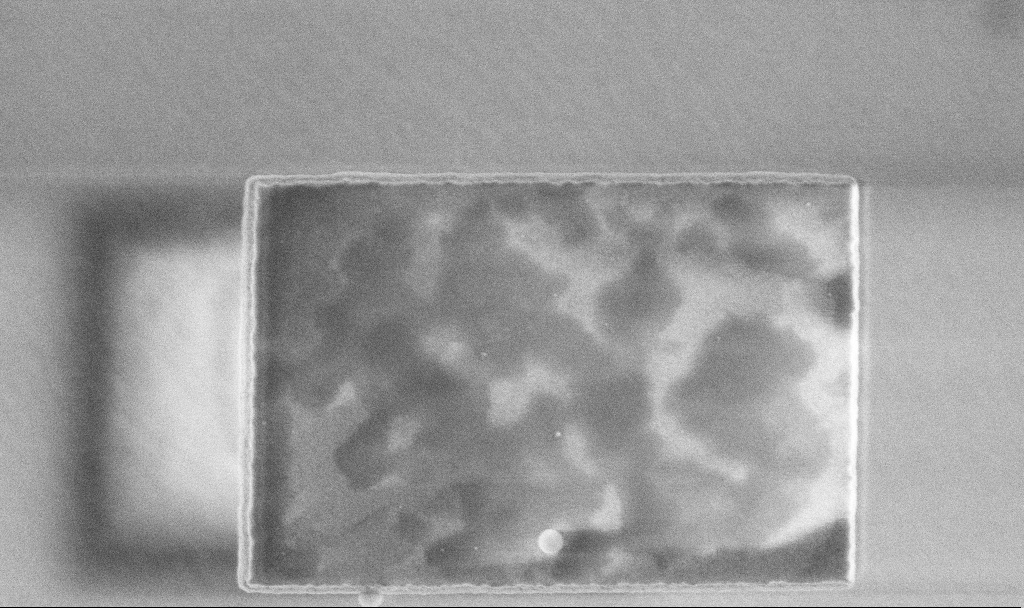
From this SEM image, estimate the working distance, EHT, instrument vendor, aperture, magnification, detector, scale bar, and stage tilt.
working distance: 3.3 mm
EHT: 3 kV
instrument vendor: Zeiss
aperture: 30 µm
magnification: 50.49 K X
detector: InLens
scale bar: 1000 nm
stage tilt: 0°